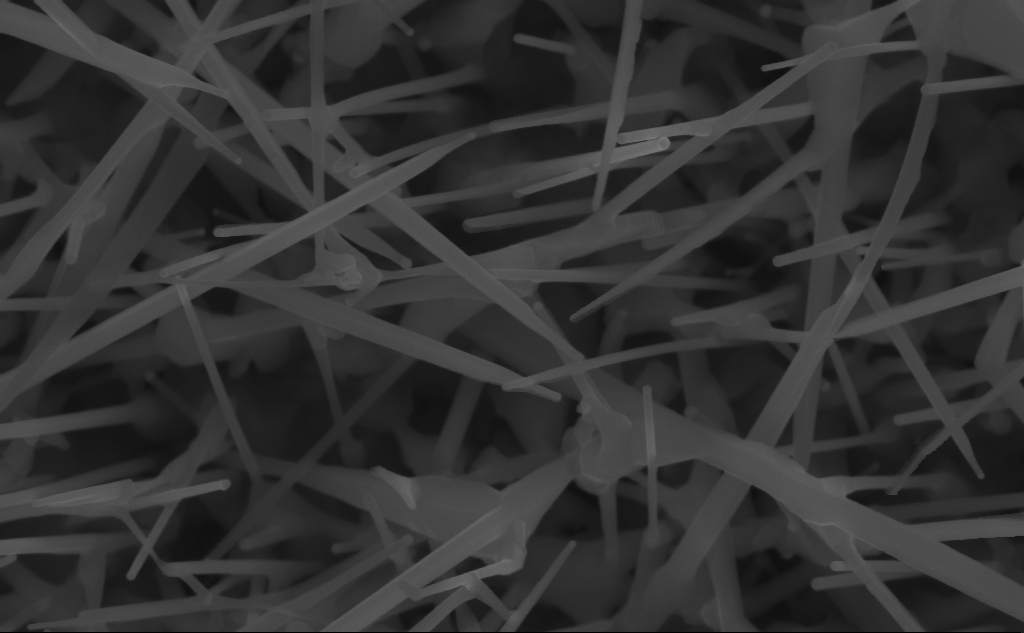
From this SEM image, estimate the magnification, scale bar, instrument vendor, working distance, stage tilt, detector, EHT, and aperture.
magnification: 110.96 K X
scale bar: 200 nm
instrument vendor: Zeiss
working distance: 7 mm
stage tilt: -0°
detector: InLens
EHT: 10 kV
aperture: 30 µm